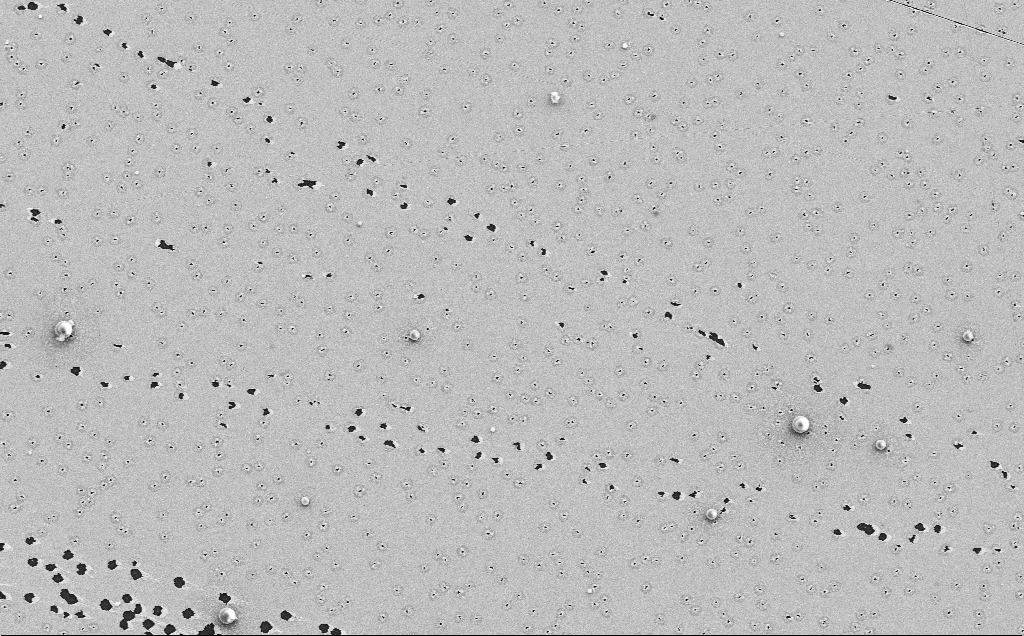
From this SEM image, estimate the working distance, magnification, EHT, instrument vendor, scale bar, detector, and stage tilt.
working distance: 12 mm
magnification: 4.2 K X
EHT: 5 kV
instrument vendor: Zeiss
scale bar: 10000 nm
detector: SE2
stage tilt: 0°